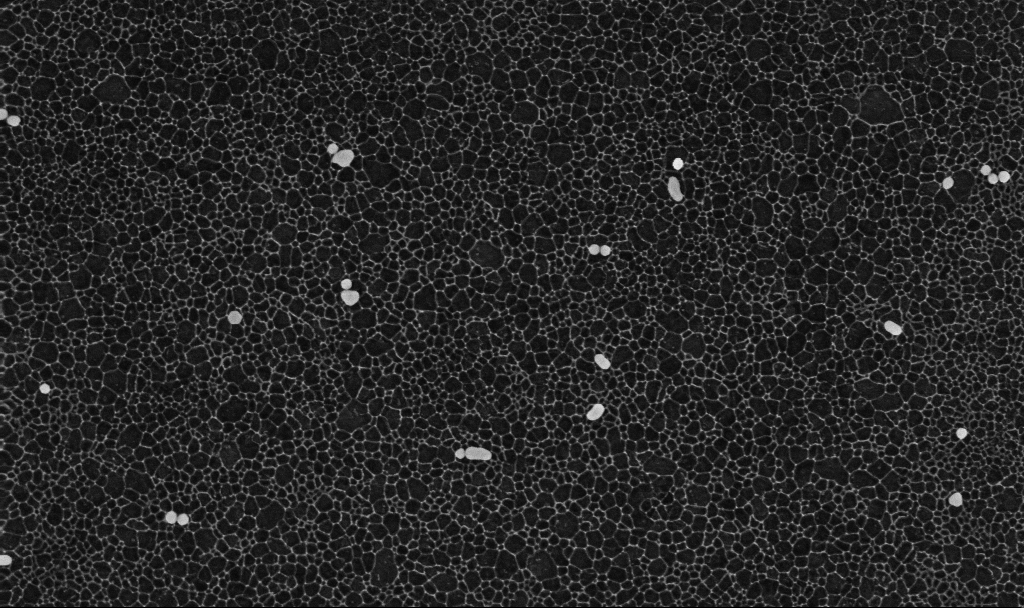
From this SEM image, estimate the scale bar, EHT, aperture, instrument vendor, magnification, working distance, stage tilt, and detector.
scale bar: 1000 nm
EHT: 10 kV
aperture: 30 µm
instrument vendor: Zeiss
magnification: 70 K X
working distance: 3.4 mm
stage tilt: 0°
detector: InLens